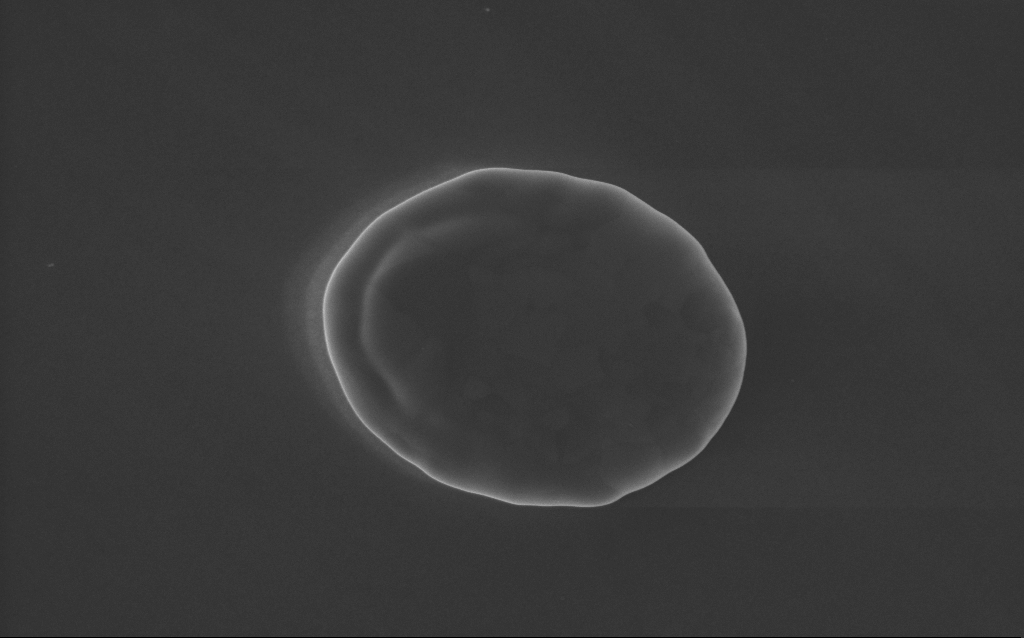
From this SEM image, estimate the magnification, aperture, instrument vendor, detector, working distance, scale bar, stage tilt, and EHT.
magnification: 53 K X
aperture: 30 µm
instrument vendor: Zeiss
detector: InLens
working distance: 3 mm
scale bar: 1000 nm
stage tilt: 0°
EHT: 5 kV